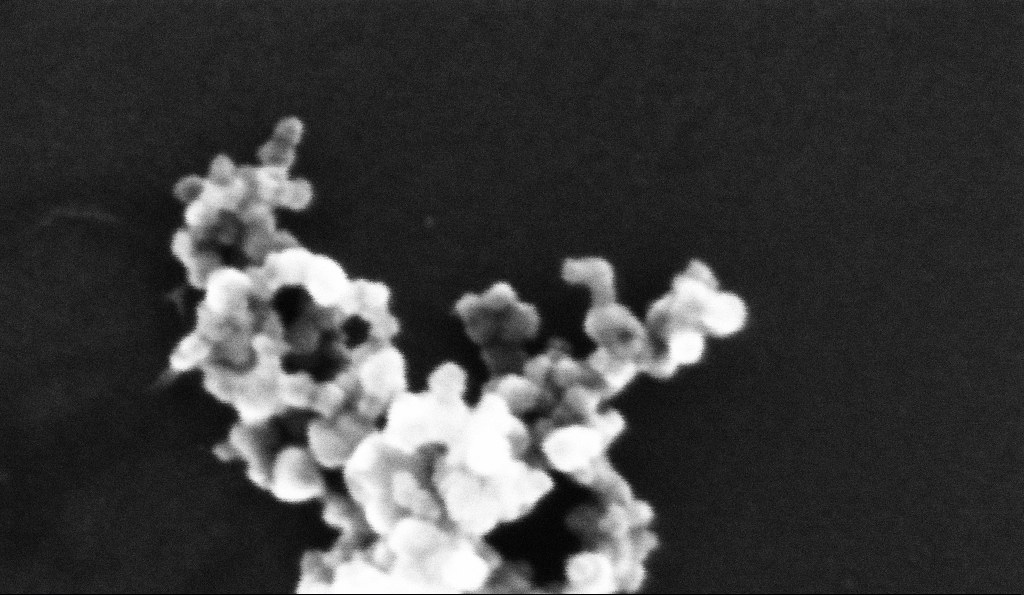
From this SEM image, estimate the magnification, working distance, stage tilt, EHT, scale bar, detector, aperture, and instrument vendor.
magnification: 744.8 K X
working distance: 5.2 mm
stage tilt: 0°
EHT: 10 kV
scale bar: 20 nm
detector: InLens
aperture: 30 µm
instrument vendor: Zeiss